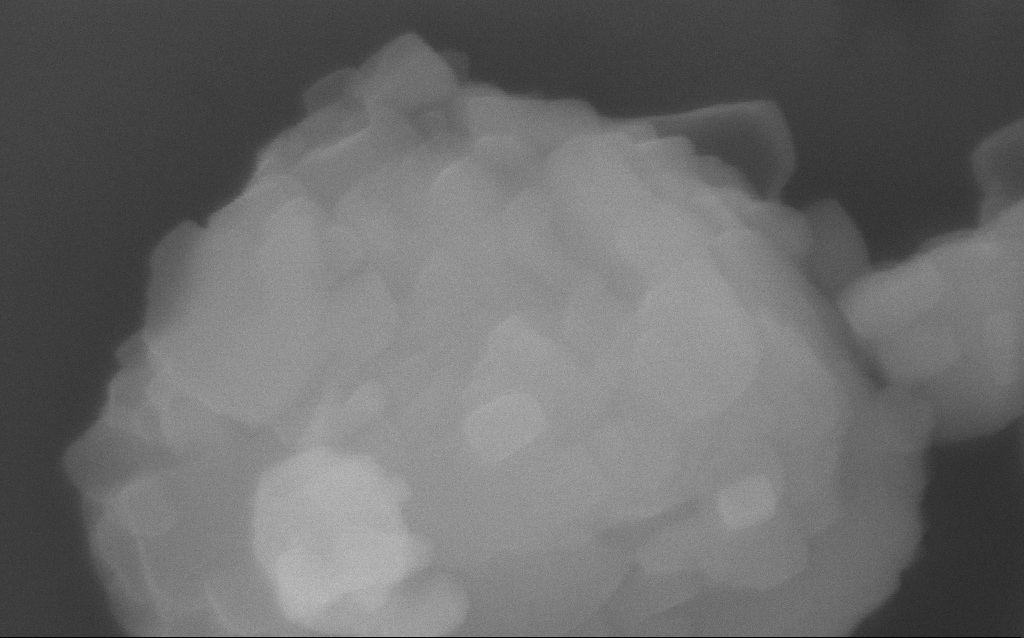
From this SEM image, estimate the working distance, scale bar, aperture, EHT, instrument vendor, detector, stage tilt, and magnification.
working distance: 3 mm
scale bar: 100 nm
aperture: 30 µm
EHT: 10 kV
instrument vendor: Zeiss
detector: InLens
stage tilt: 0°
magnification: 290.72 K X